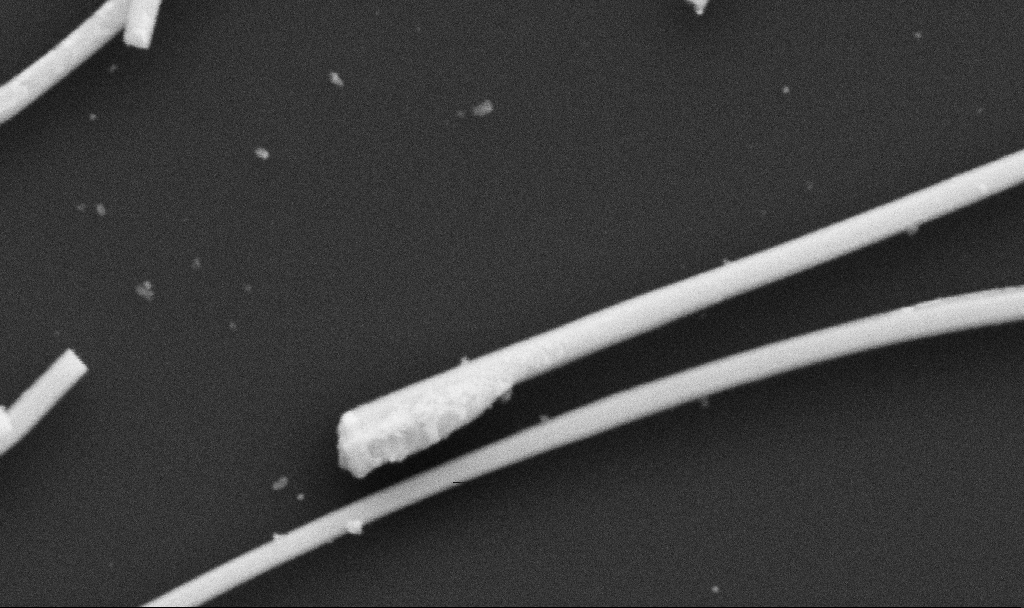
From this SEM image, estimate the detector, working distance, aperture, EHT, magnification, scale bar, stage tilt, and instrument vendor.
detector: SE2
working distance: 10.7 mm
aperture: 30 µm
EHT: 5 kV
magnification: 105.2 K X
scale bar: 200 nm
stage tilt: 0°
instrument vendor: Zeiss